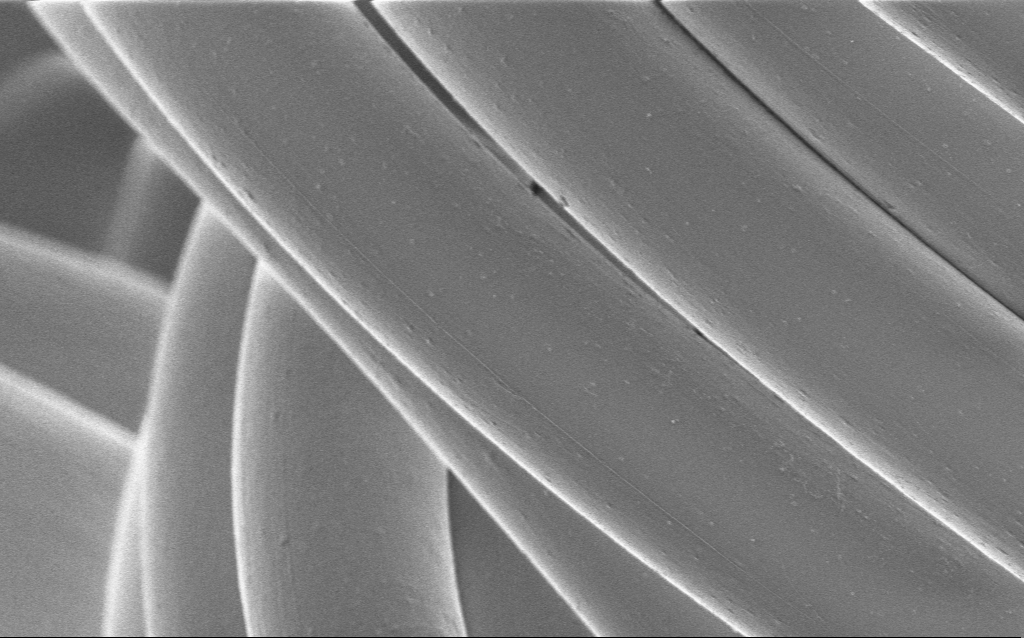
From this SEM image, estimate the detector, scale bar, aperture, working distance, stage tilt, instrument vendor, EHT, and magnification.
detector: InLens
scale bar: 20000 nm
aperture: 30 µm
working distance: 4 mm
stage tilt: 0°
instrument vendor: Zeiss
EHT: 1 kV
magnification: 3.31 K X